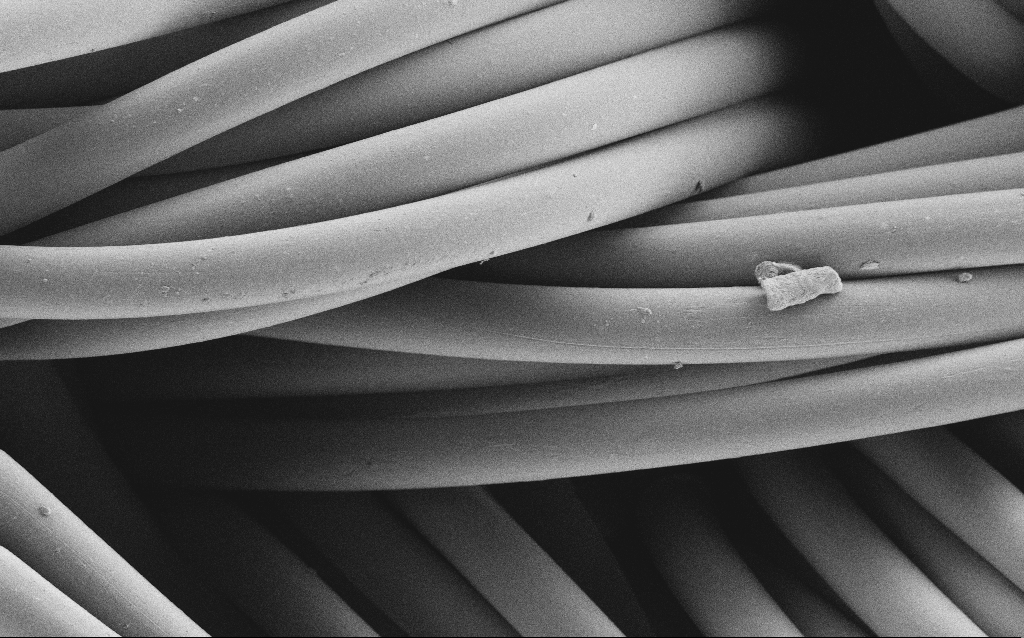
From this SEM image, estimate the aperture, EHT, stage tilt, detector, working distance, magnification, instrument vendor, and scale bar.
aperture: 30 µm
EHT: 1 kV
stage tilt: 0°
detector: SE2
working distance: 4 mm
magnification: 1.21 K X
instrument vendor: Zeiss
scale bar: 20000 nm